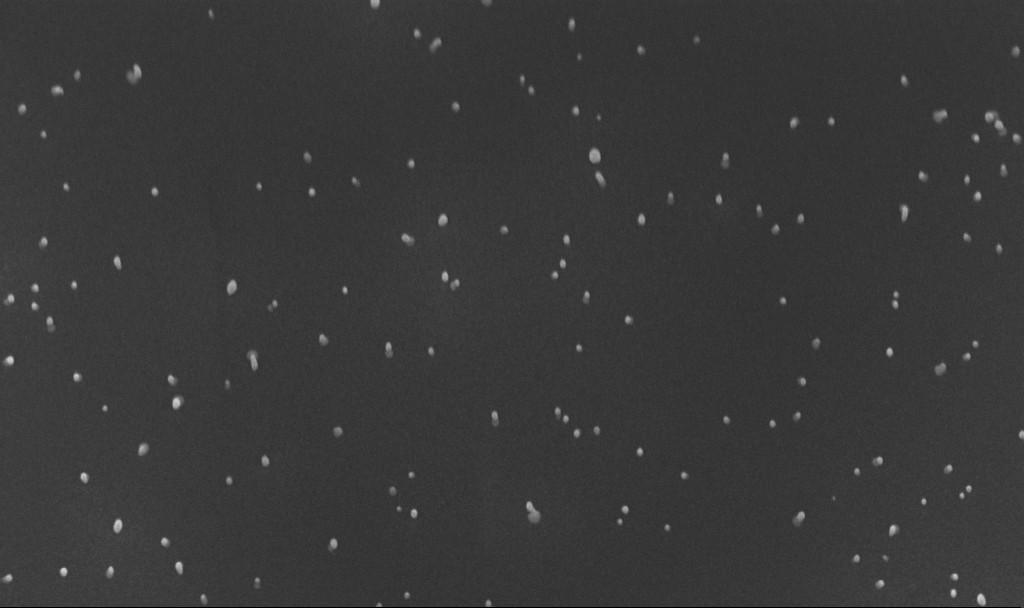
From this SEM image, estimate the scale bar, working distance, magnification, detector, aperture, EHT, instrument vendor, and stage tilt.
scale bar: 200 nm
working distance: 4.9 mm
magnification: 100 K X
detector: InLens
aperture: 30 µm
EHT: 10 kV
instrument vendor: Zeiss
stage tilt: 45°